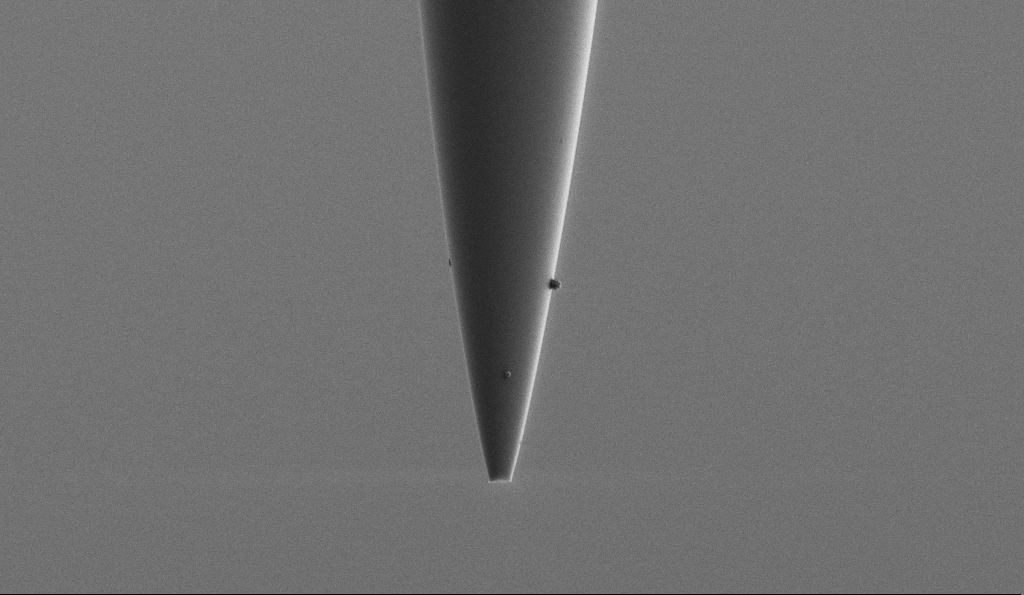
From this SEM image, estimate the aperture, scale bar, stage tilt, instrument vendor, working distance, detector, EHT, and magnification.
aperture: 30 µm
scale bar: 2000 nm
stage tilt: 0°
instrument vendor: Zeiss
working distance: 6.5 mm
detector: SE2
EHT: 1 kV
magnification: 10 K X